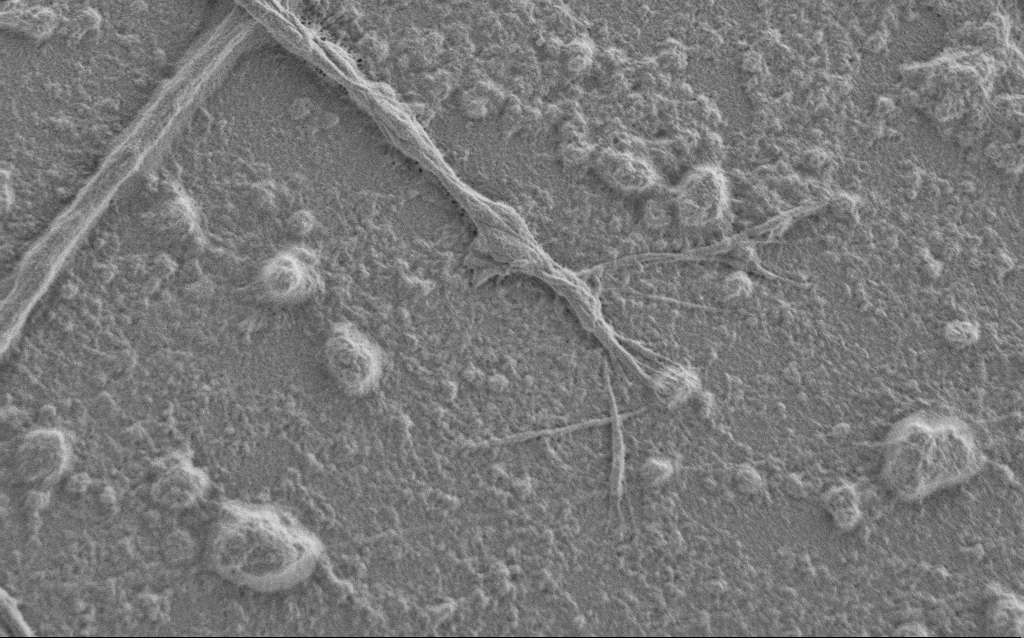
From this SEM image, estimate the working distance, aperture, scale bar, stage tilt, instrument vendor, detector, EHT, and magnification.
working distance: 6 mm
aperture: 30 µm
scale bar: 2000 nm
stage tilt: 0°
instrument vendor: Zeiss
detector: SE2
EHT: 1 kV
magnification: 7.5 K X